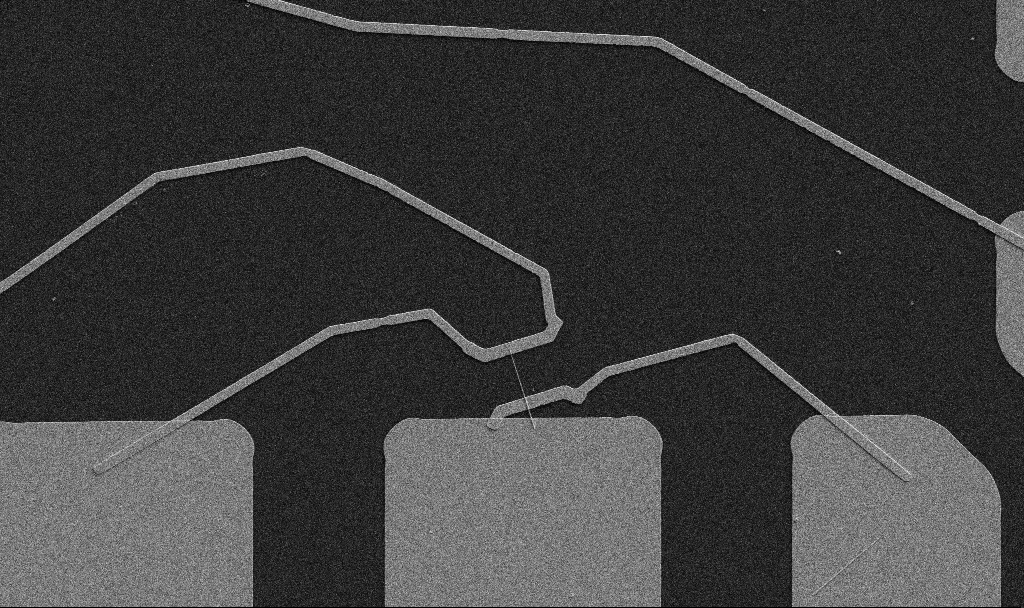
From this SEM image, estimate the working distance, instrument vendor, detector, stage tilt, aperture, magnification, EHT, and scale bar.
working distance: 10.7 mm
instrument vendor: Zeiss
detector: SE2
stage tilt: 0°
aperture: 30 µm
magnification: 5 K X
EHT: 5 kV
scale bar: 10000 nm